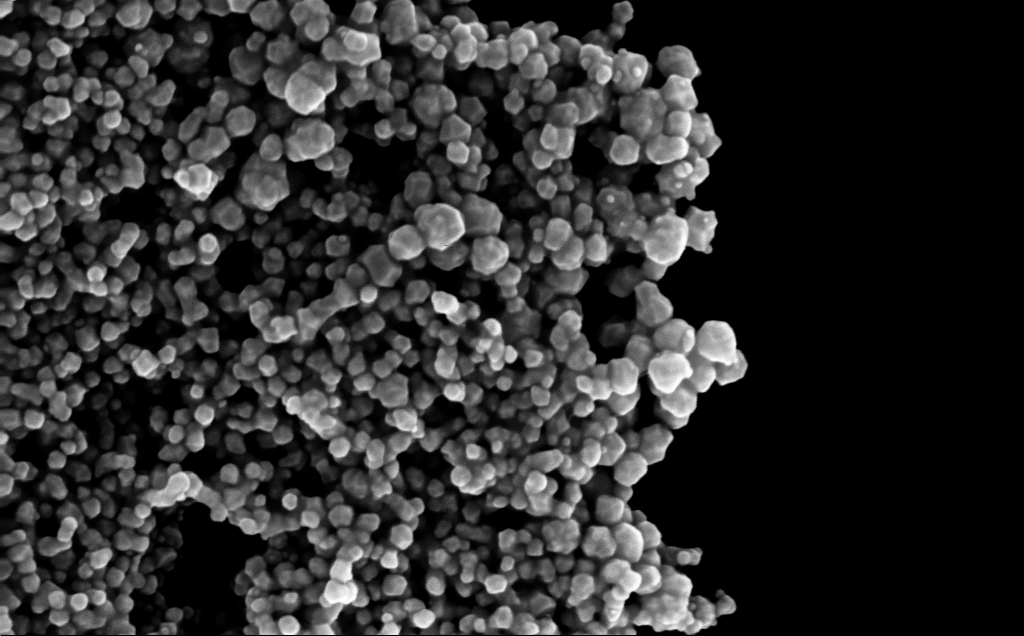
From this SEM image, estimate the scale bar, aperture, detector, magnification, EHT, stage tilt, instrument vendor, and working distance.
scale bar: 200 nm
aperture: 30 µm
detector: InLens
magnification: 165.6 K X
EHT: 10 kV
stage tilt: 0°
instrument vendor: Zeiss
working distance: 4 mm